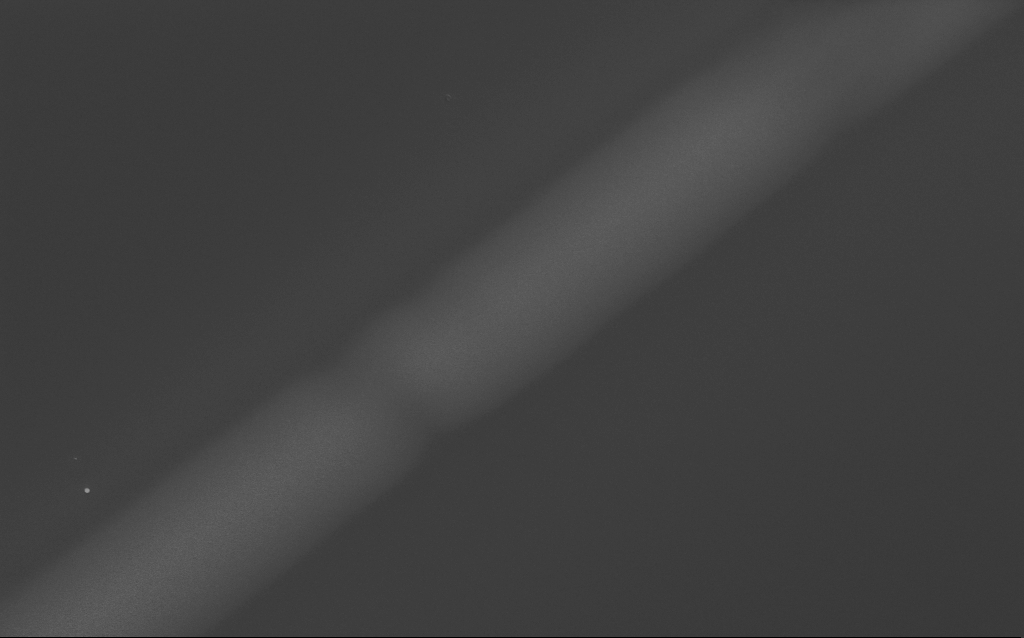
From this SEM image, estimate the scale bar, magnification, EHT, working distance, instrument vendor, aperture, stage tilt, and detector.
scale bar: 2000 nm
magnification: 11.78 K X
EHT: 3 kV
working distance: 3 mm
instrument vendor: Zeiss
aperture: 30 µm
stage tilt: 0°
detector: InLens